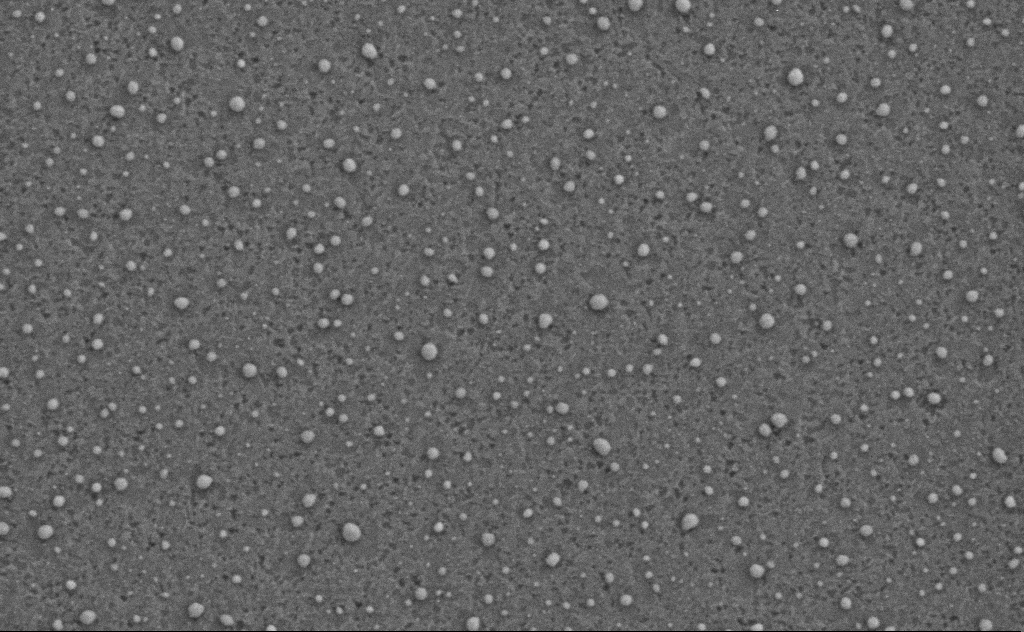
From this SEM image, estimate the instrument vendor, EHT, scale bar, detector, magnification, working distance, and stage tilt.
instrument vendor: Zeiss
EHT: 3 kV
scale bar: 200 nm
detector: SE2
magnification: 80 K X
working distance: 4 mm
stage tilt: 0°